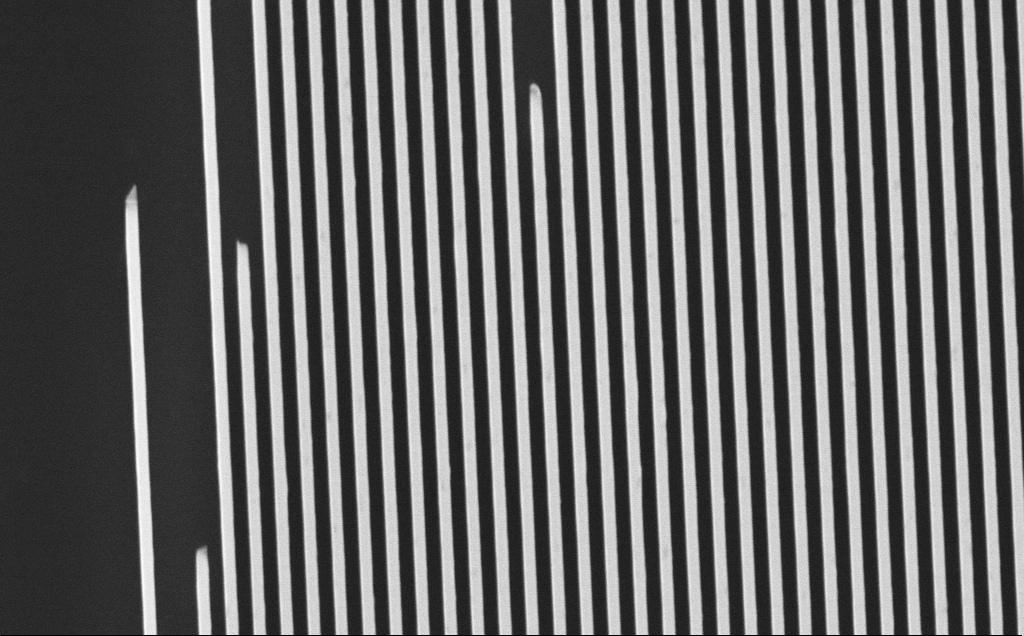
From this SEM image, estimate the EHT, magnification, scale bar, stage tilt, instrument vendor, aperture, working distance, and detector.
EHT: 10 kV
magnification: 20.19 K X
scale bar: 2000 nm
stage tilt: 25°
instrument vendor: Zeiss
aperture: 30 µm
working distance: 4 mm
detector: InLens